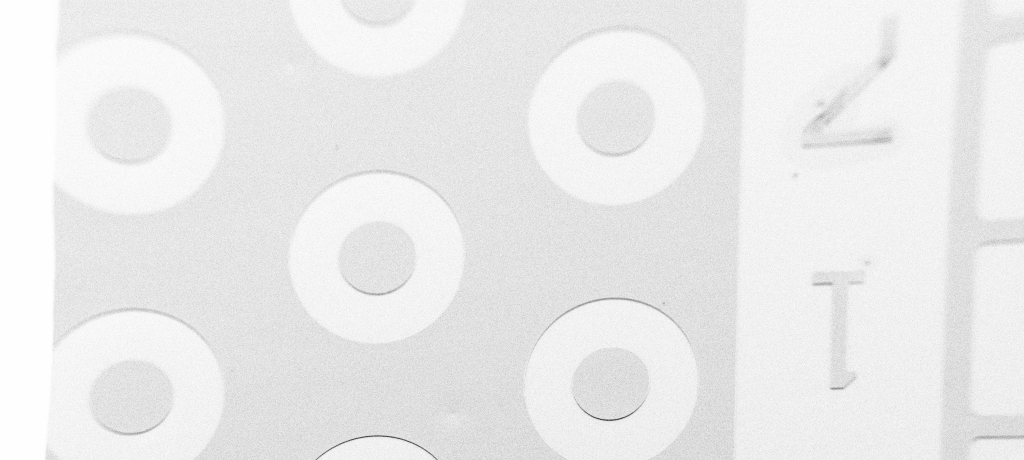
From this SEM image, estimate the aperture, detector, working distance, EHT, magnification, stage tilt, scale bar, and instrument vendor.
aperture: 30 µm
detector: SE2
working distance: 6 mm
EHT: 1.7 kV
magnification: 0.177 K X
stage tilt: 45°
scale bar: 100000 nm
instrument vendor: Zeiss